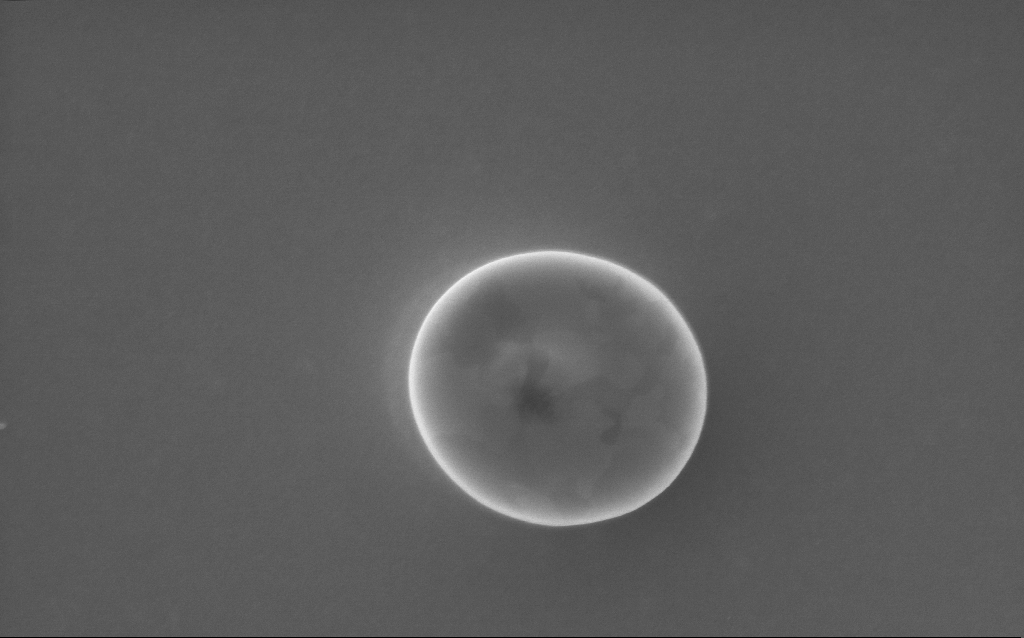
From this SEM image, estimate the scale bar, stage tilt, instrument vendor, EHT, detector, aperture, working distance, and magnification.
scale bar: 1000 nm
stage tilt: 0°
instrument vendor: Zeiss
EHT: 5 kV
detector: InLens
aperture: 30 µm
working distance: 2 mm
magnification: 63.37 K X